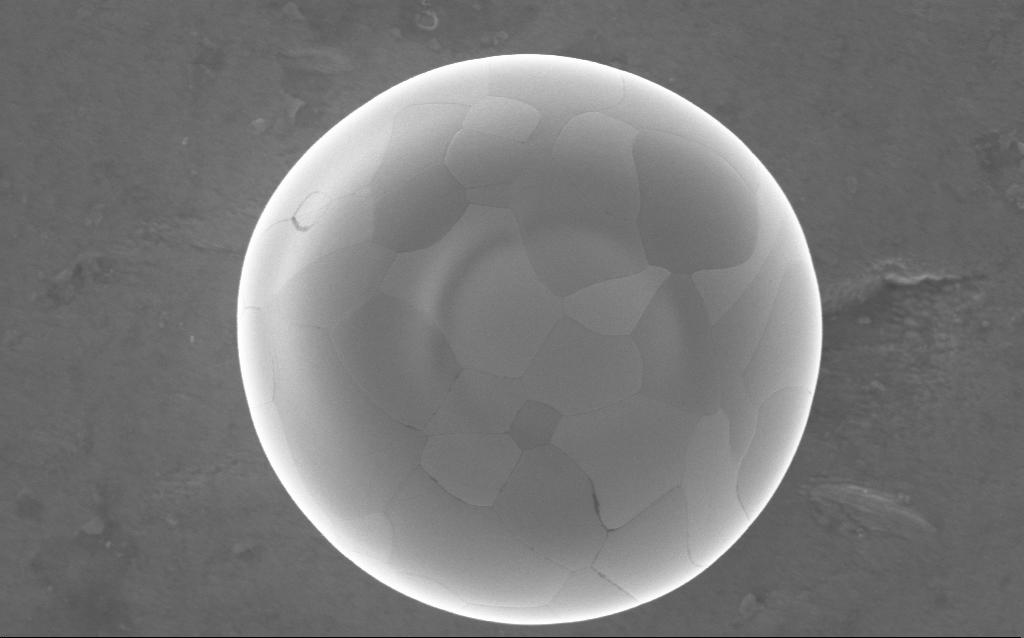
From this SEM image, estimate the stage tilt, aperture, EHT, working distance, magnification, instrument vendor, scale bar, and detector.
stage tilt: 0°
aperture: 30 µm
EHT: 5 kV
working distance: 3 mm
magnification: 64 K X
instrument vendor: Zeiss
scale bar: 1000 nm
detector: InLens